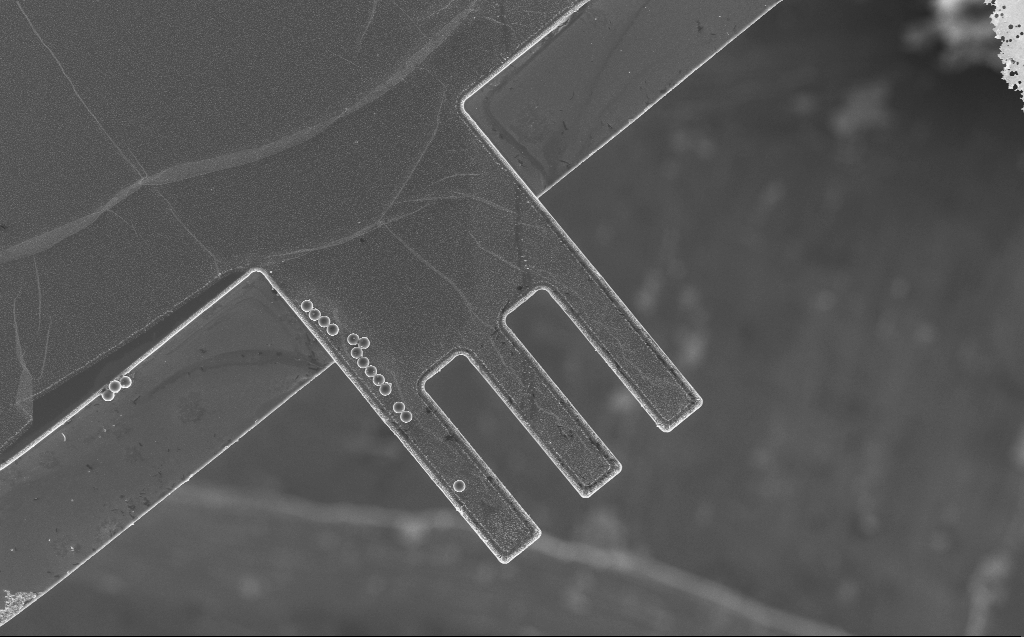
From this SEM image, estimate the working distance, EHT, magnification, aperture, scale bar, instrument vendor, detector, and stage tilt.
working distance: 5 mm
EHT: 10 kV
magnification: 0.959 K X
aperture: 30 µm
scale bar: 20000 nm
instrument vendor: Zeiss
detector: InLens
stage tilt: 0°